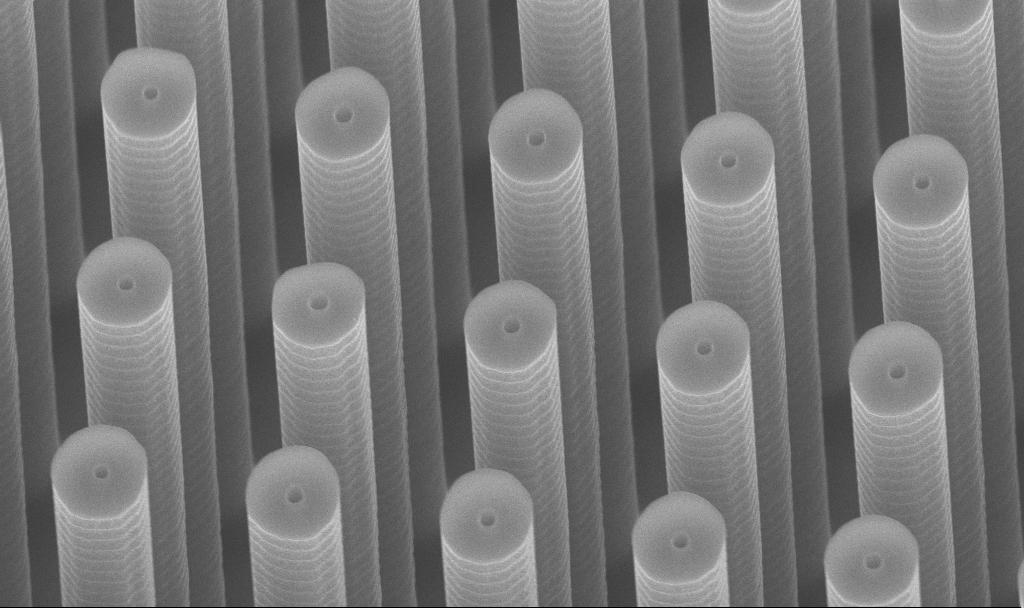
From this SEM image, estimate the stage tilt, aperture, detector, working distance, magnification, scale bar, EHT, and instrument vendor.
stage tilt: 45°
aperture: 30 µm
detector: InLens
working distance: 8.3 mm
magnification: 18.05 K X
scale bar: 2000 nm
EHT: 10 kV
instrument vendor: Zeiss